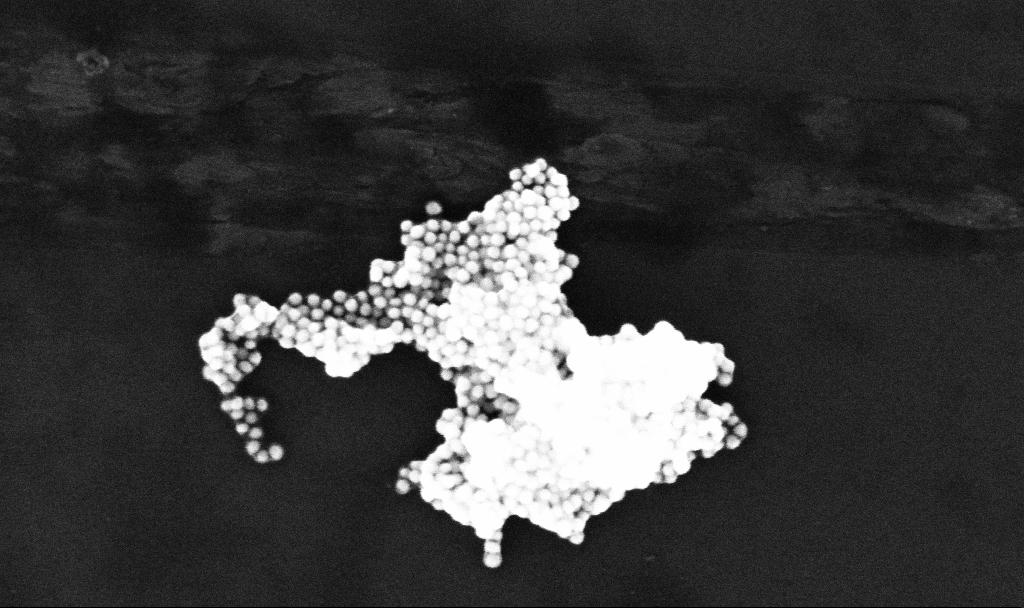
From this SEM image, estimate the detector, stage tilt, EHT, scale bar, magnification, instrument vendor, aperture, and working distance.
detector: InLens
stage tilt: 0°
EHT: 10 kV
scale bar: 100 nm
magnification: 229.08 K X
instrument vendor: Zeiss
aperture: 30 µm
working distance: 3.4 mm